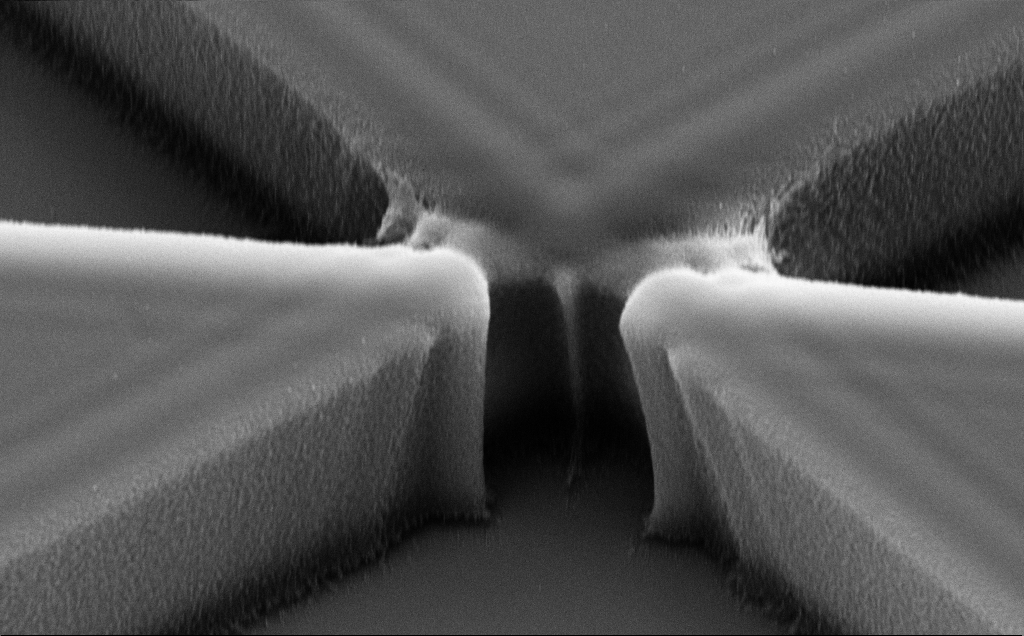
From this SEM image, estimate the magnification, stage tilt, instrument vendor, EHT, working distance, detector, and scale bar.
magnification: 14.61 K X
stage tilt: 35.3°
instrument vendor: Zeiss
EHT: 10 kV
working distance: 11 mm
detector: SE2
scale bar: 2000 nm